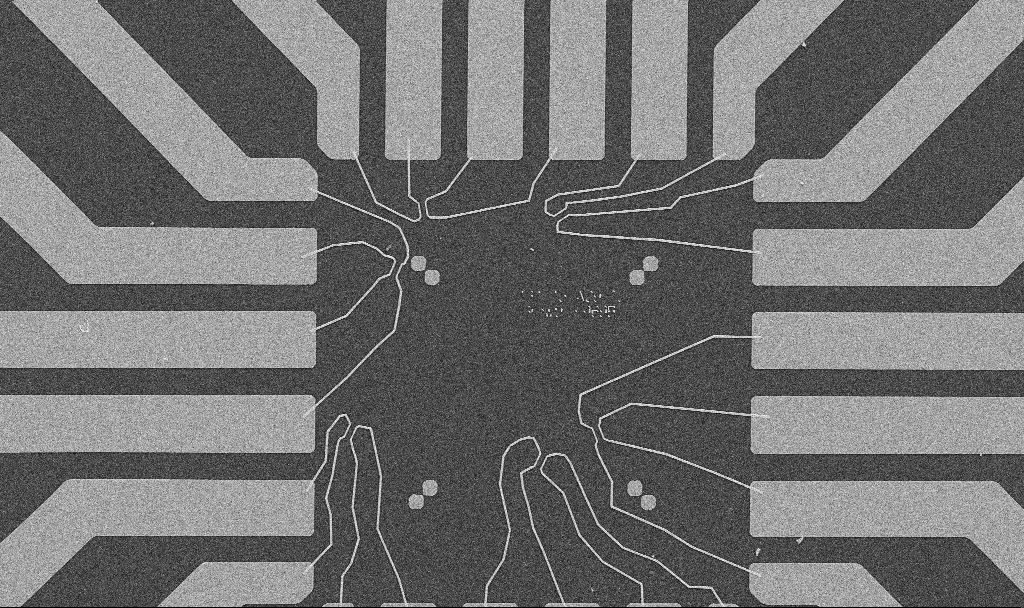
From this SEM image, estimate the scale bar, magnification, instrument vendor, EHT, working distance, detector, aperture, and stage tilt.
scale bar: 20000 nm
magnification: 1 K X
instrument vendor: Zeiss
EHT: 10 kV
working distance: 10.7 mm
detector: SE2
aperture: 30 µm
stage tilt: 0°